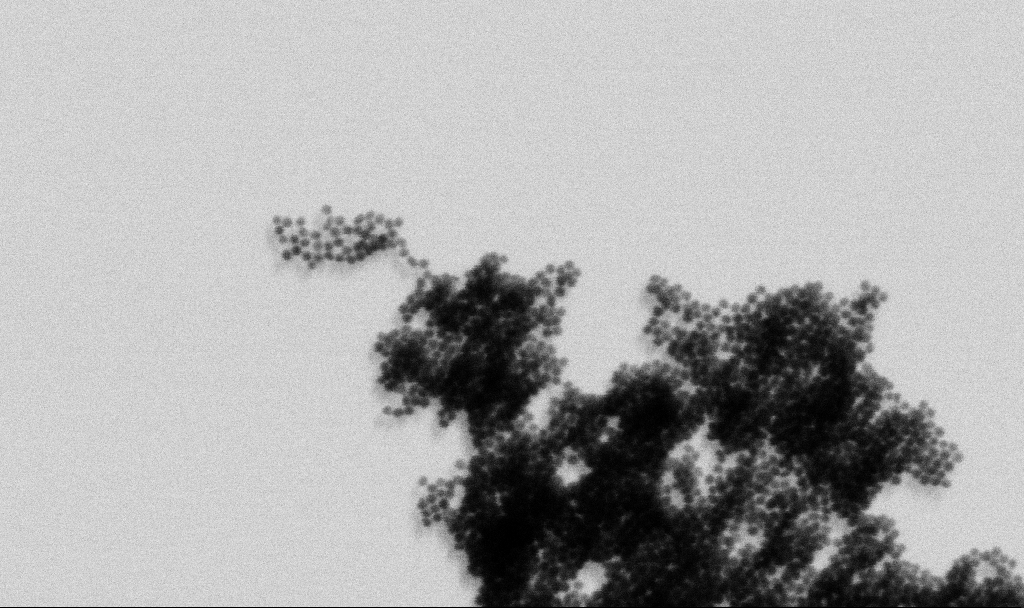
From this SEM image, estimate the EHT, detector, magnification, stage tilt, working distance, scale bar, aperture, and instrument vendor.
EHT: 4 kV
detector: SE2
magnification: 200 K X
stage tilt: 0°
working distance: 6 mm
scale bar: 100 nm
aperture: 30 µm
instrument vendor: Zeiss